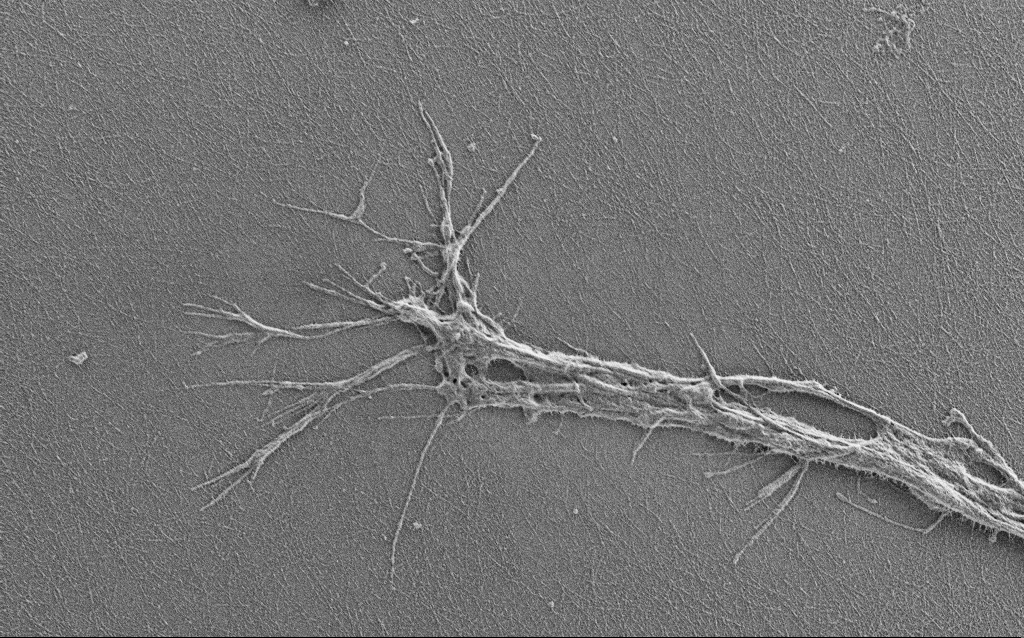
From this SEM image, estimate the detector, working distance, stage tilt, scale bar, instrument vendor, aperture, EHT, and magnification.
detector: SE2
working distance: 4 mm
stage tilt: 0°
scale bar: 2000 nm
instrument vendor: Zeiss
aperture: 30 µm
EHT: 1 kV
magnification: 7.5 K X